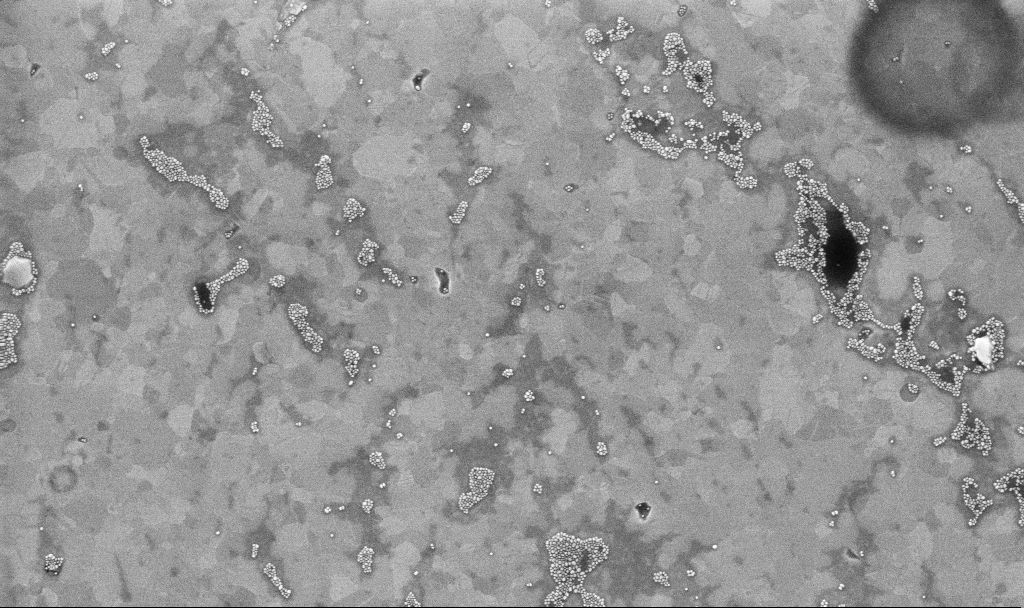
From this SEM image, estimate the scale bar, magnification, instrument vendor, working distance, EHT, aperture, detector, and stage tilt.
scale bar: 1000 nm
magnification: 50.18 K X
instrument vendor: Zeiss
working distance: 3.1 mm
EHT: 10 kV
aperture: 30 µm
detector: InLens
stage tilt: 0°